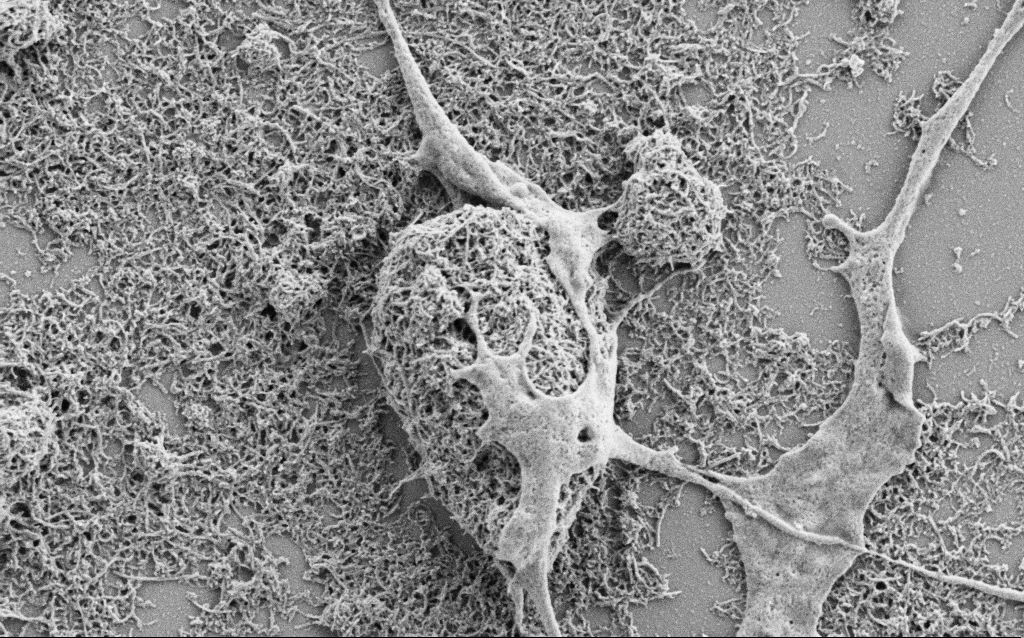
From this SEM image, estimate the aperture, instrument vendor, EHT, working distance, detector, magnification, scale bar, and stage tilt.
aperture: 30 µm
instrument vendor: Zeiss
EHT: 1.5 kV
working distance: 6.9 mm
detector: SE2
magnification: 20 K X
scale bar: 1000 nm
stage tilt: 0°